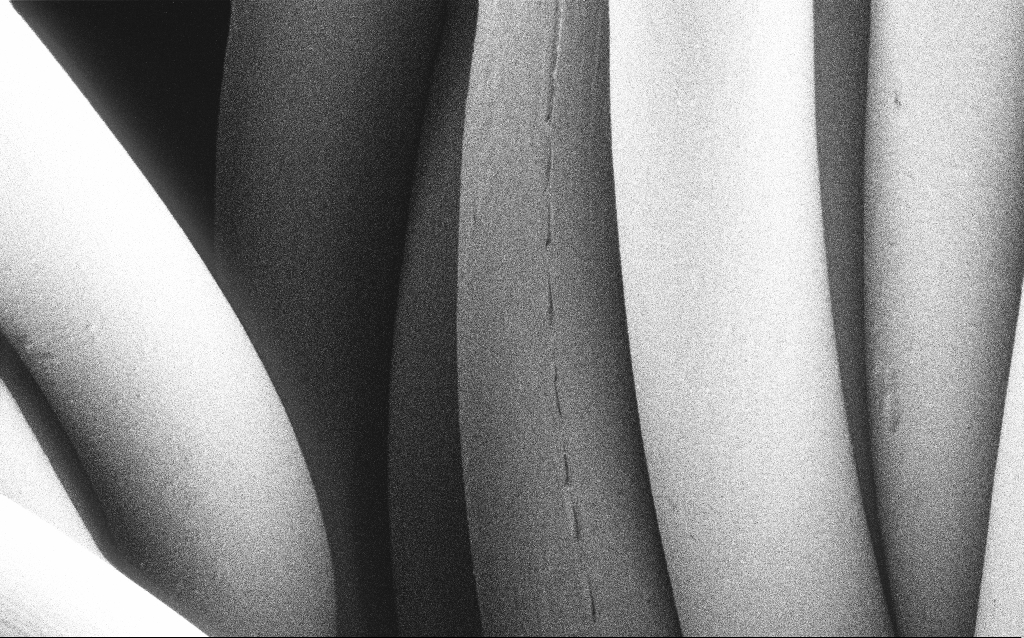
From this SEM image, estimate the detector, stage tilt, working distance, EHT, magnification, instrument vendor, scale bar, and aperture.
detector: SE2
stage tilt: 0°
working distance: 4 mm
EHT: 1 kV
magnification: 3.2 K X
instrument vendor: Zeiss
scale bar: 20000 nm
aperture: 30 µm